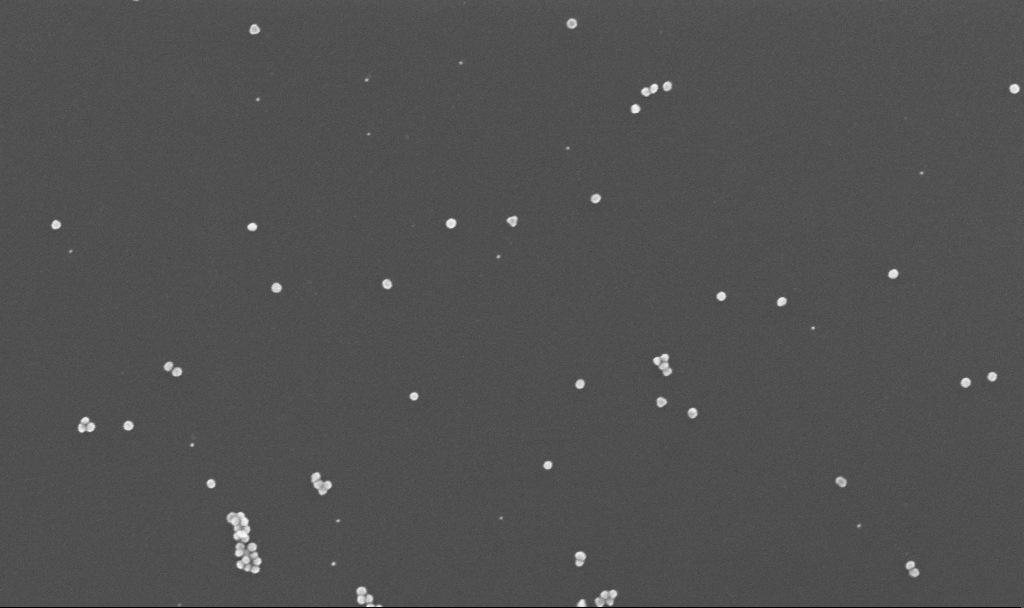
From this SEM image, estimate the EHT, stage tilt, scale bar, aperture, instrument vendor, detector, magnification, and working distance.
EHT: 10 kV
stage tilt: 0°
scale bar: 200 nm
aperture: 30 µm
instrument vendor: Zeiss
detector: InLens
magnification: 150 K X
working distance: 3.4 mm